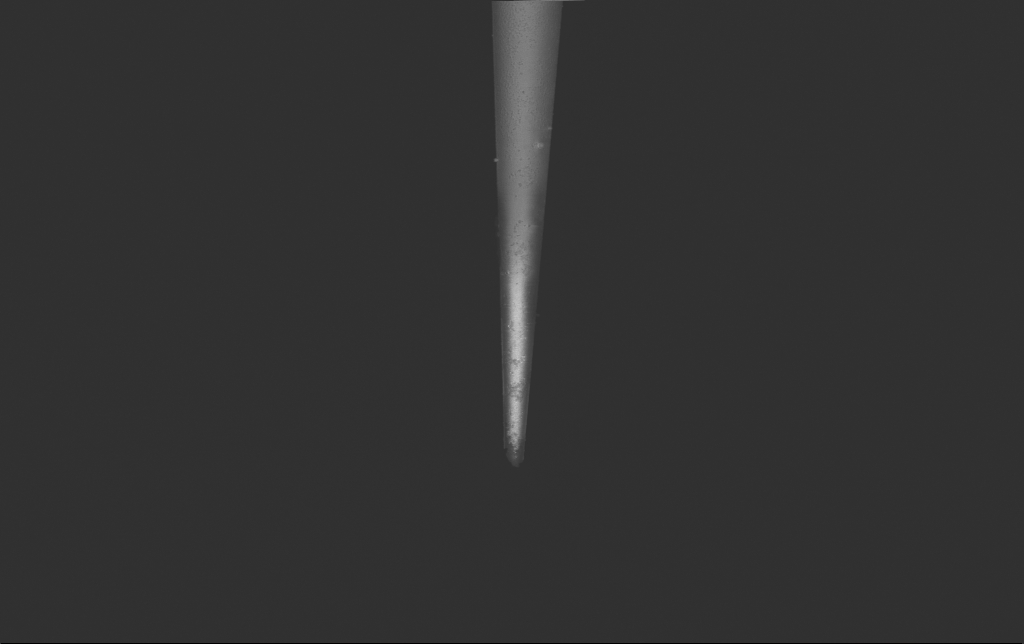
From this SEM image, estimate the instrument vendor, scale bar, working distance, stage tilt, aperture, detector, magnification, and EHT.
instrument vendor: Zeiss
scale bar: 2000 nm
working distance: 6 mm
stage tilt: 0°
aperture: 30 µm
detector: InLens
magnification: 7.5 K X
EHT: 2 kV